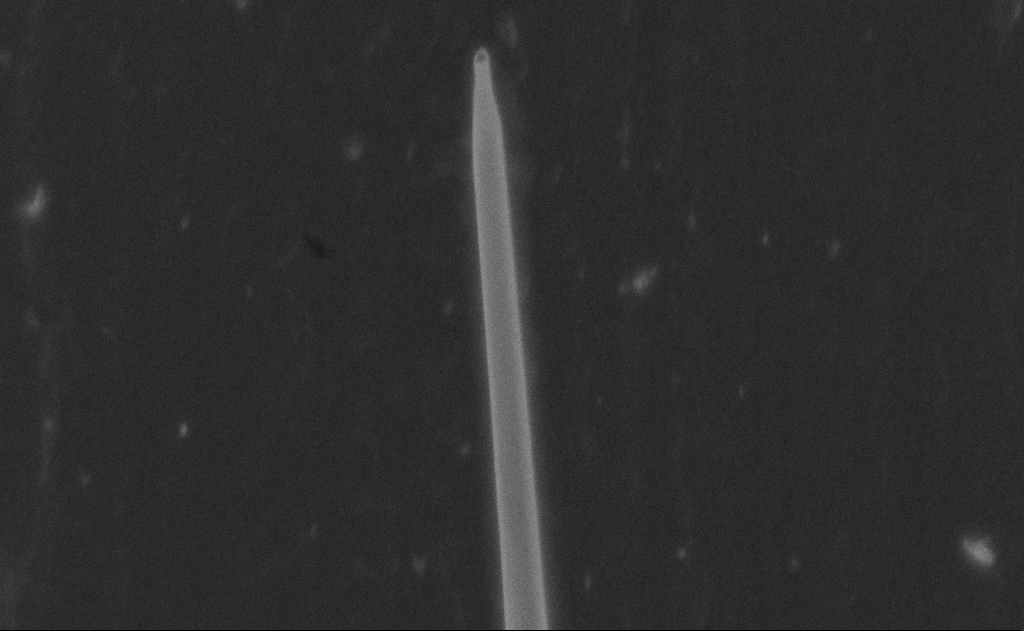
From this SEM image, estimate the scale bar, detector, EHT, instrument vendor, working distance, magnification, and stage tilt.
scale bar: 200 nm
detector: SE2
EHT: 20 kV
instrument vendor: Zeiss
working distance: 9 mm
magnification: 125.87 K X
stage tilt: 0°